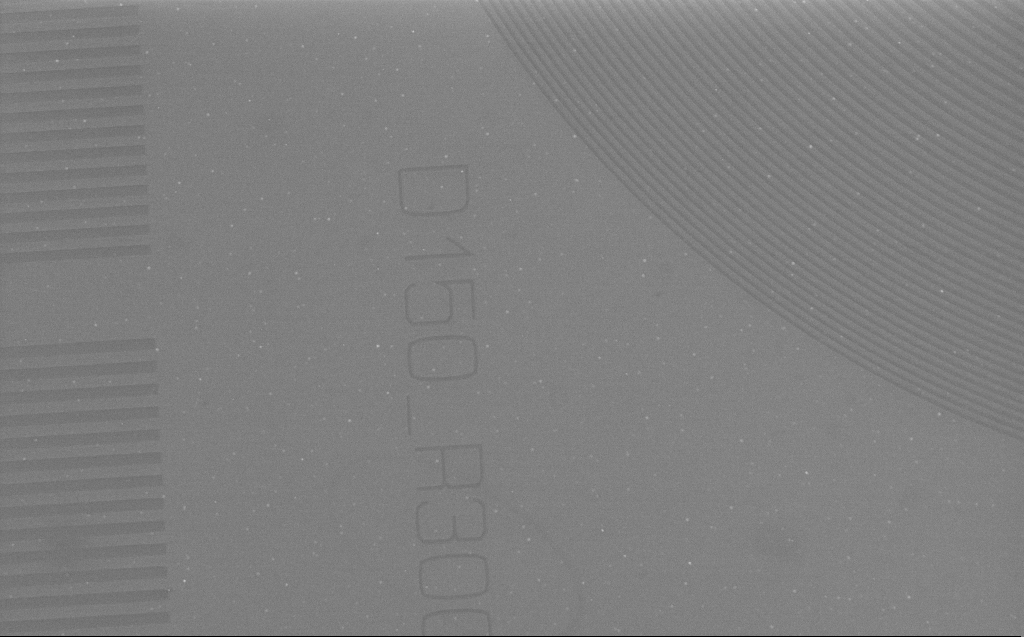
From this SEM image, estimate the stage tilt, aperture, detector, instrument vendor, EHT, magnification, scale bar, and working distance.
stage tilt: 0°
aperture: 30 µm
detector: InLens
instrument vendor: Zeiss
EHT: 1 kV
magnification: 5.3 K X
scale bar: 10000 nm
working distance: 4 mm